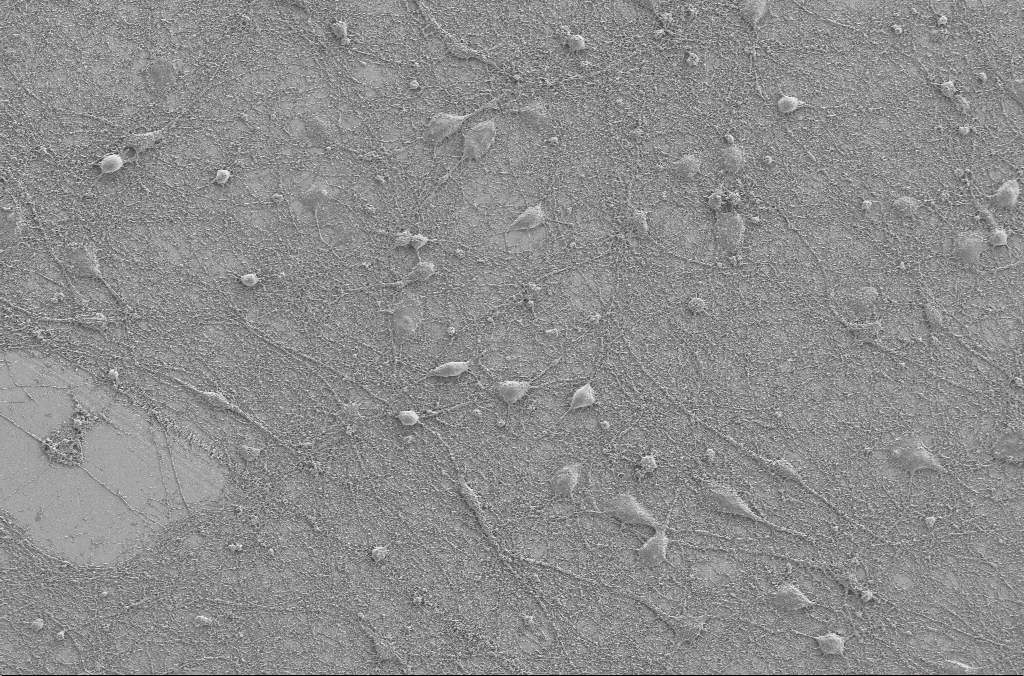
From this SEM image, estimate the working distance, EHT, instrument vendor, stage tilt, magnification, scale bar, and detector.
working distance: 4 mm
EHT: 2 kV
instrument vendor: Zeiss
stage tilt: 0°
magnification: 1 K X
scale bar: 20000 nm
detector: SE2